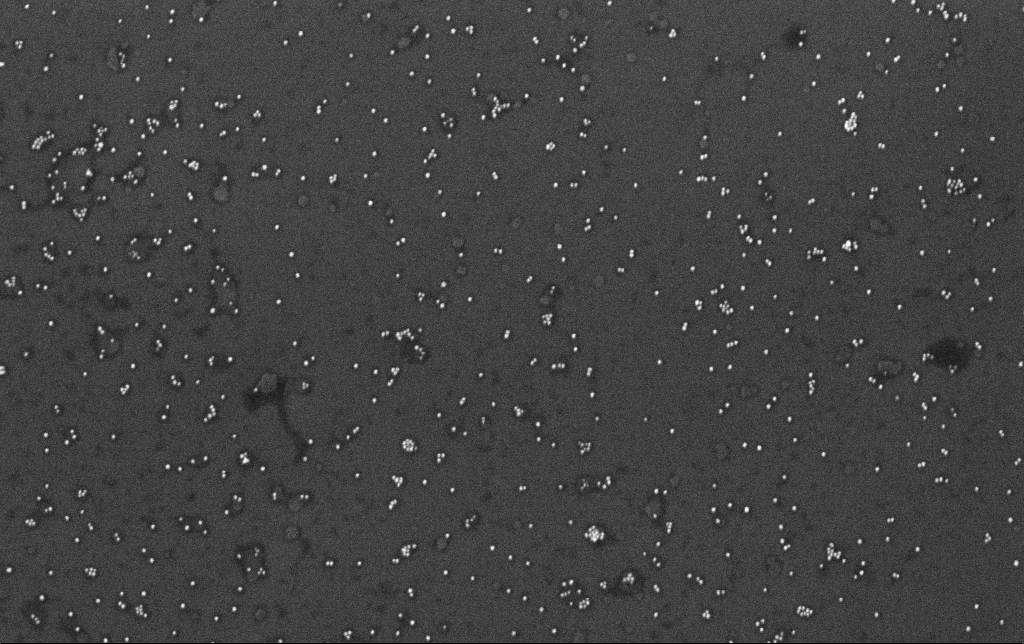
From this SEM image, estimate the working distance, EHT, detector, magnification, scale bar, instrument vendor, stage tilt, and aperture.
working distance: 3.4 mm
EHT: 10 kV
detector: InLens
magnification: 100 K X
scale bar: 200 nm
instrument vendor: Zeiss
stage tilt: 0°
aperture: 30 µm